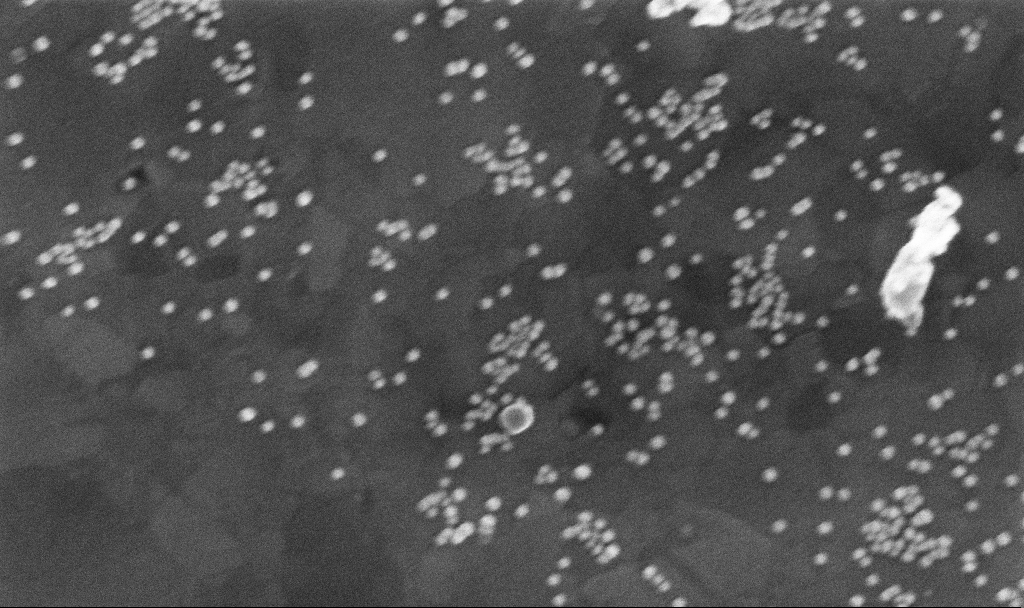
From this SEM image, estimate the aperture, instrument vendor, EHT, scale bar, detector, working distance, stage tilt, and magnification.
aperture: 30 µm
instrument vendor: Zeiss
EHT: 10 kV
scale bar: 200 nm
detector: InLens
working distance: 3.7 mm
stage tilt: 0°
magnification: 200 K X